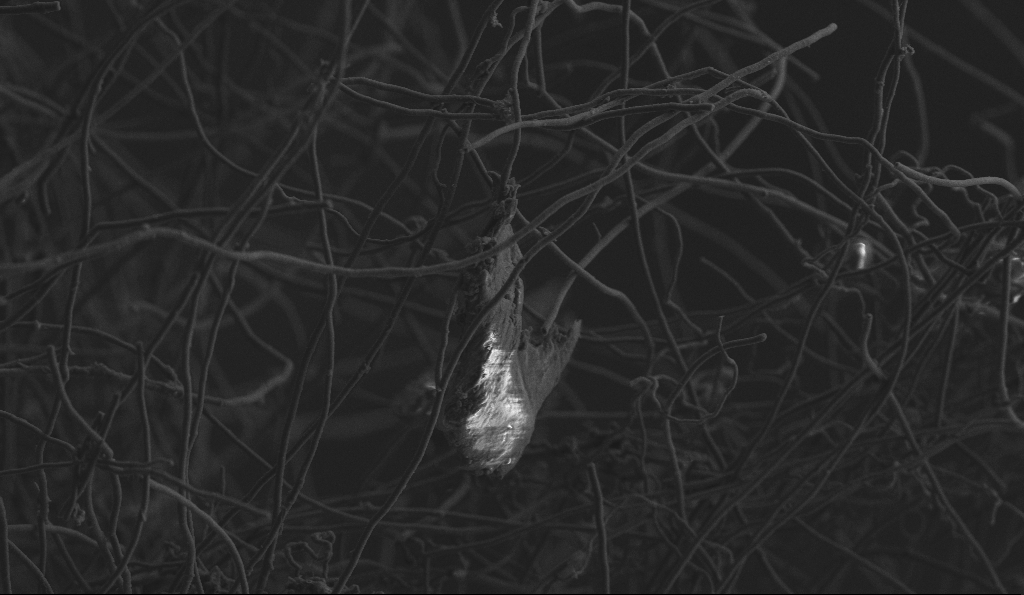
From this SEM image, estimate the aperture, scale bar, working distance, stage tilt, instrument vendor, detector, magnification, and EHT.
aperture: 30 µm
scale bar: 10000 nm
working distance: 5.9 mm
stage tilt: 0°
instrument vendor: Zeiss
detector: SE2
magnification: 5 K X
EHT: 3 kV